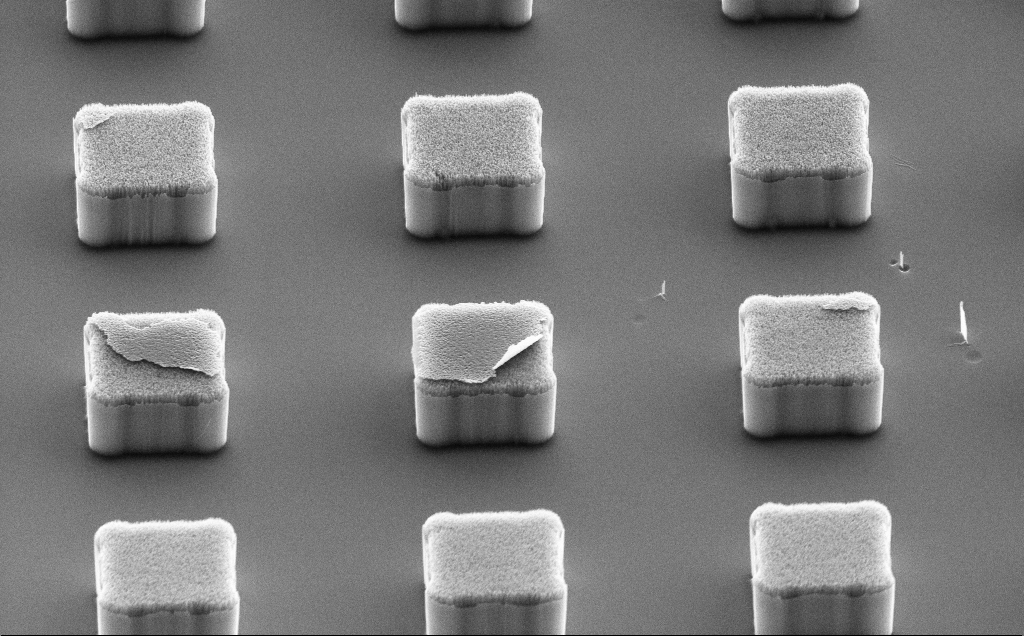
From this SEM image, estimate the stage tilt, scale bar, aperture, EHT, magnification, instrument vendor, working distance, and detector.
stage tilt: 50.6°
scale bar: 10000 nm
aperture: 30 µm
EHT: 10 kV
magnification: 6 K X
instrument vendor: Zeiss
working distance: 13 mm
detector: SE2